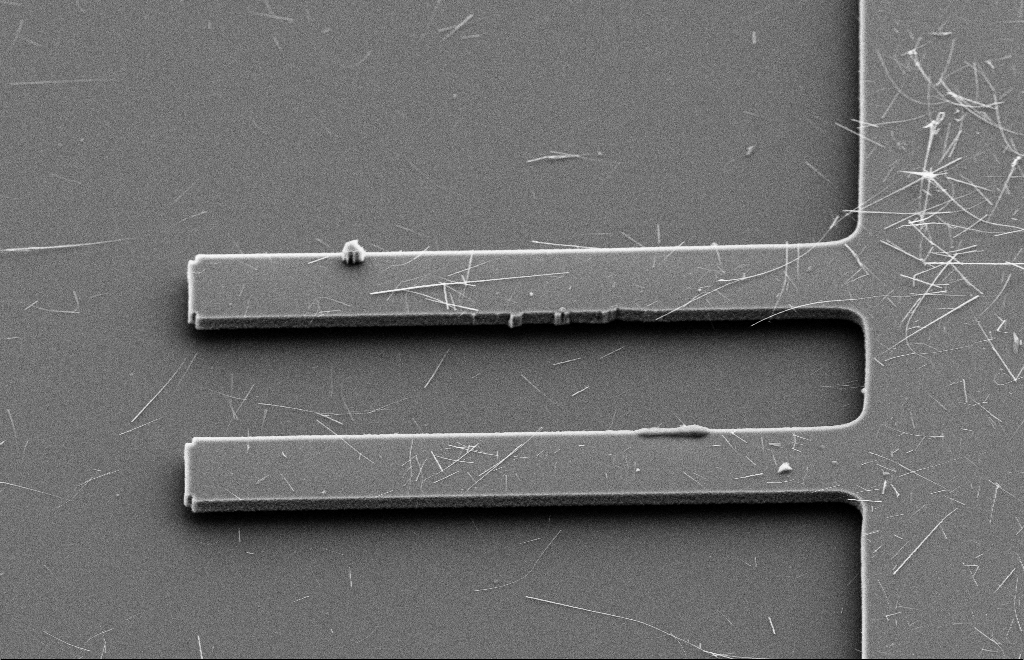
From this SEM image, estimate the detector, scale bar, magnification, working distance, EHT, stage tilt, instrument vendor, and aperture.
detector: SE2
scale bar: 10000 nm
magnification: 2.5 K X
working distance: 9 mm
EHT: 10 kV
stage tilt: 39.8°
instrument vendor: Zeiss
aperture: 20 µm